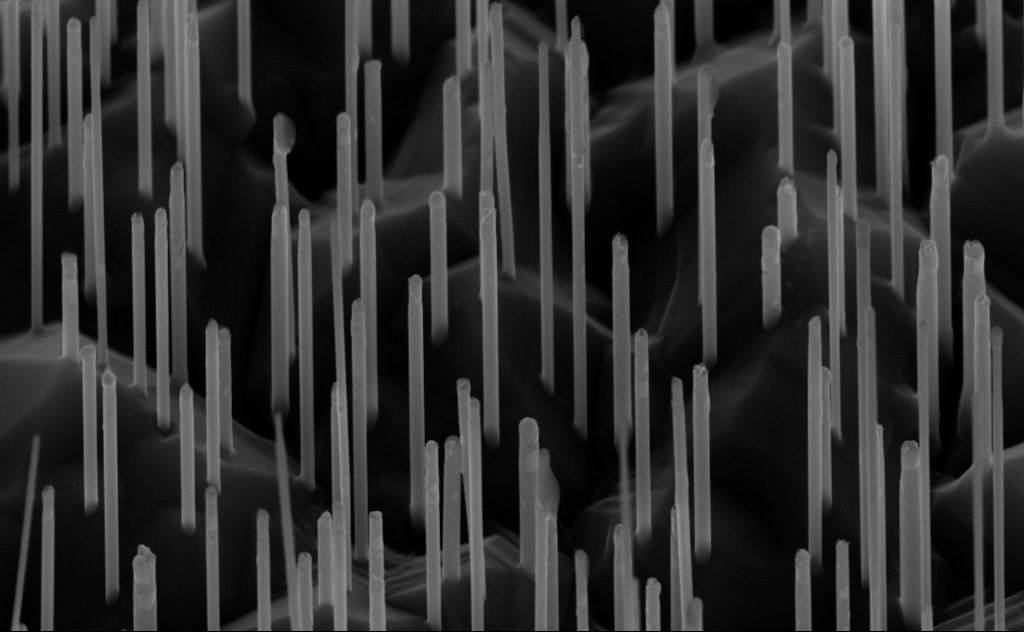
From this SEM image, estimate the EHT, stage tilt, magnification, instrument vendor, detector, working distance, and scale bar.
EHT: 10 kV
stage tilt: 45°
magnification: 80 K X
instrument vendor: Zeiss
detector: InLens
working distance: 6 mm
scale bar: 200 nm